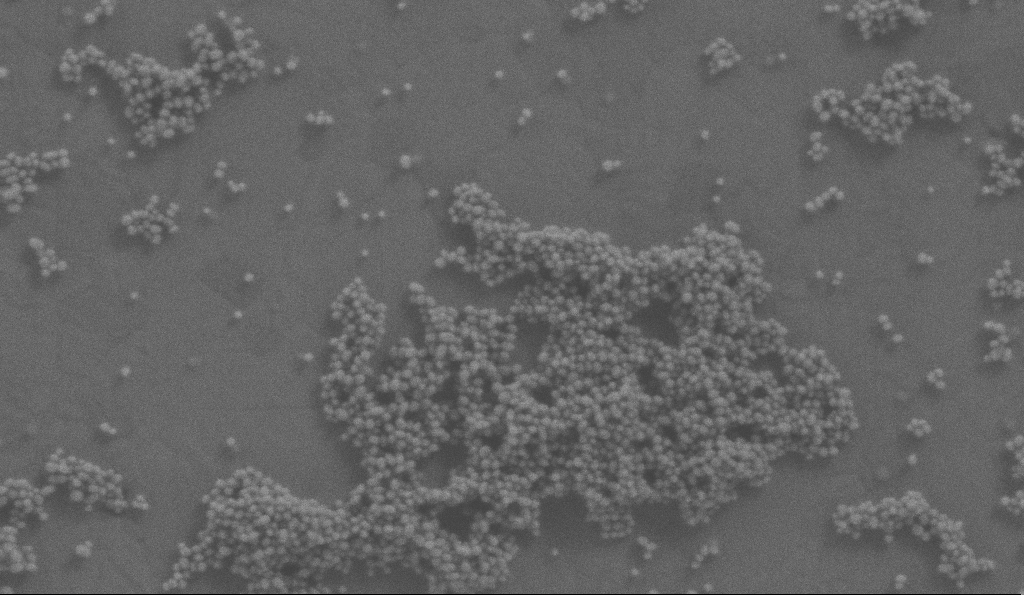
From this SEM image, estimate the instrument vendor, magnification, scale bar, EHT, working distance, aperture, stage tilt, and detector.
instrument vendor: Zeiss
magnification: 150 K X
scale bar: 100 nm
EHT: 2 kV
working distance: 3.2 mm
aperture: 30 µm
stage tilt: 0°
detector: SE2